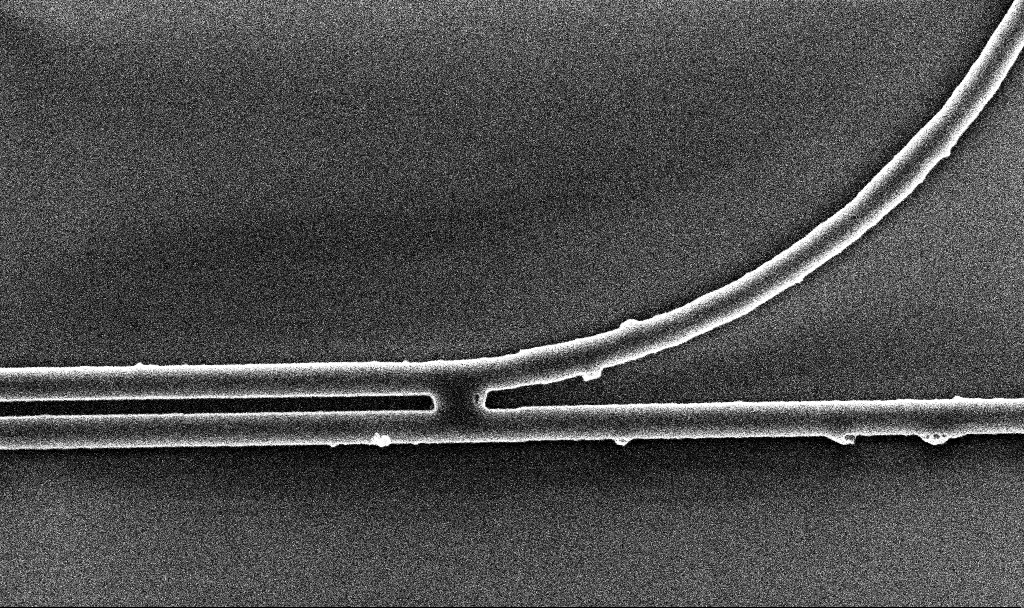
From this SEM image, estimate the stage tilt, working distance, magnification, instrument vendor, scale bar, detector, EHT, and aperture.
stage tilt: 0°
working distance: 5.2 mm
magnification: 26.25 K X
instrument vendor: Zeiss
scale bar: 2000 nm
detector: InLens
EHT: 5 kV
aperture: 30 µm